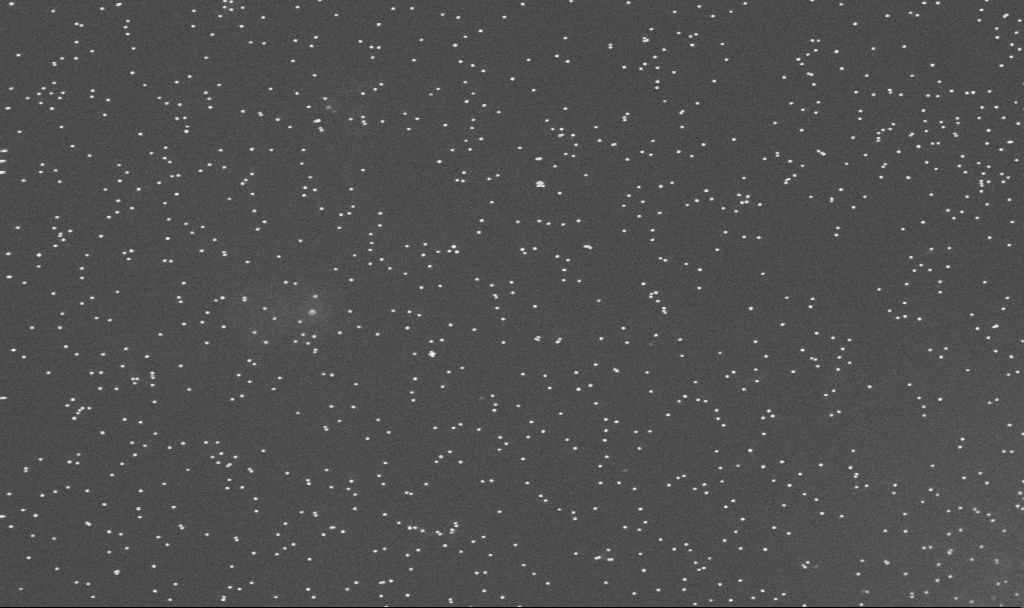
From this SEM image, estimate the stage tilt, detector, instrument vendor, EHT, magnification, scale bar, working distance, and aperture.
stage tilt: -0°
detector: InLens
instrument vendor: Zeiss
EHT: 10 kV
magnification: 100 K X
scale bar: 200 nm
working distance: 3.3 mm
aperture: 30 µm